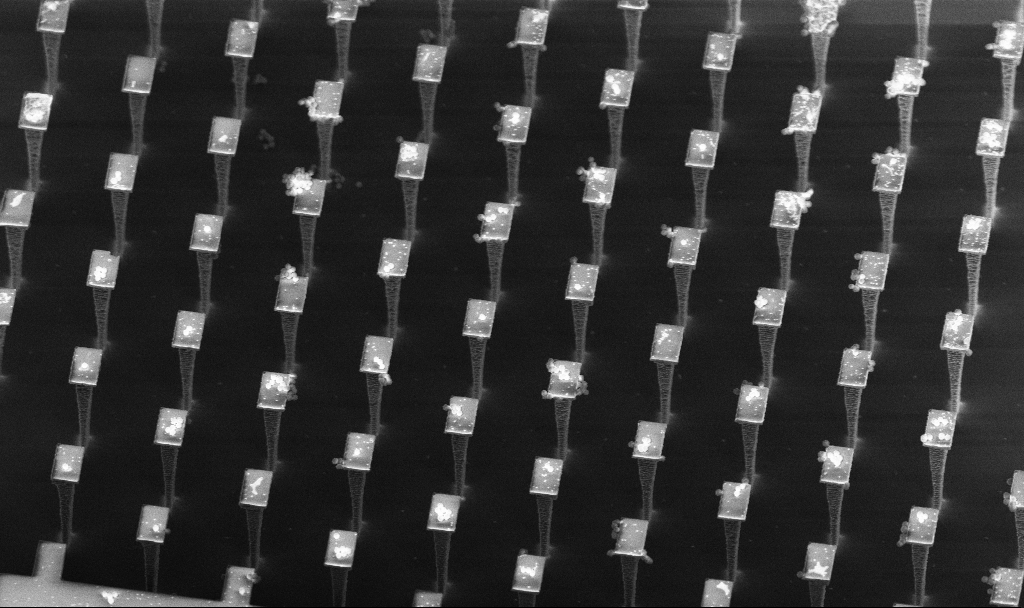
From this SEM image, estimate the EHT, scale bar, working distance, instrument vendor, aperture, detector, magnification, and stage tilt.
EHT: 5 kV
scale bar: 10000 nm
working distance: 5 mm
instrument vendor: Zeiss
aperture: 30 µm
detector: InLens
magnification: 3.48 K X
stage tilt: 30°